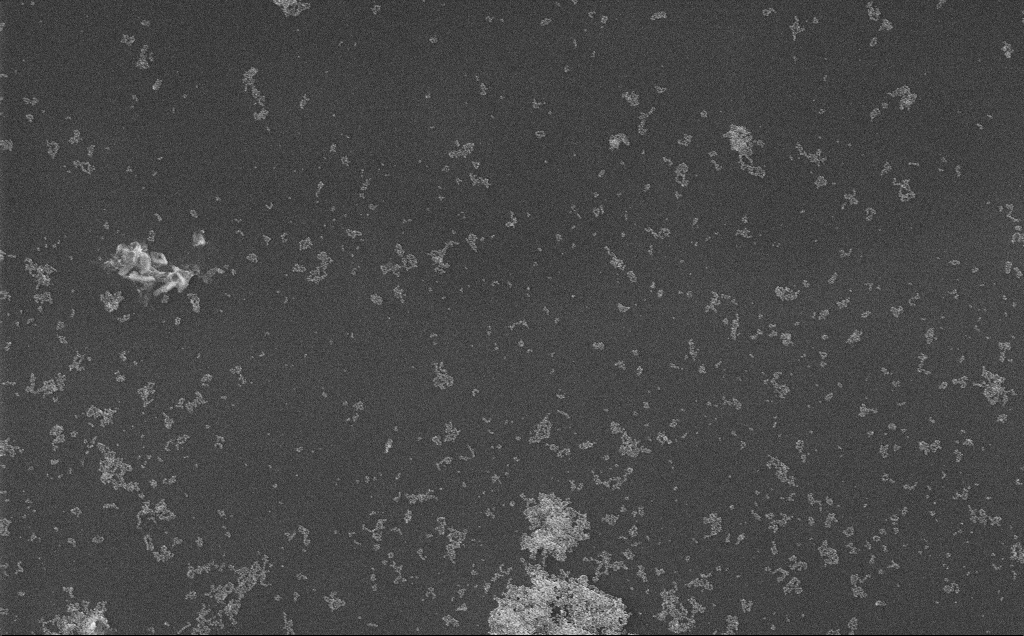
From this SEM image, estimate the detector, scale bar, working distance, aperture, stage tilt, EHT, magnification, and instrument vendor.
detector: InLens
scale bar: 2000 nm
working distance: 3.7 mm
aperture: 30 µm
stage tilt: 0°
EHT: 10 kV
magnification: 29.94 K X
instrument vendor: Zeiss